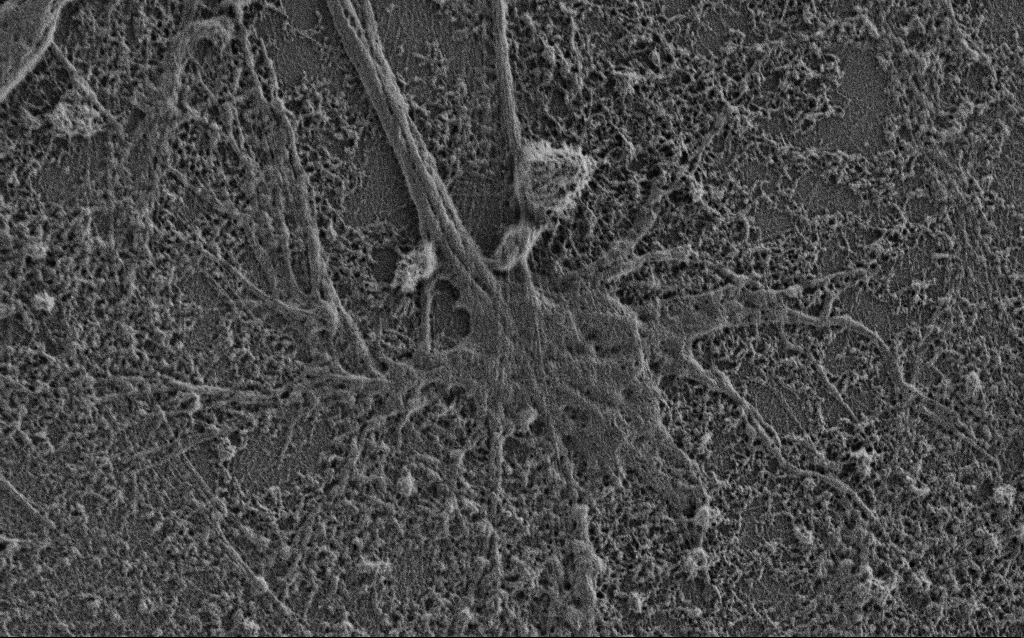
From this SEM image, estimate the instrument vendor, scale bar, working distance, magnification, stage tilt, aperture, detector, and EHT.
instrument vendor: Zeiss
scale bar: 2000 nm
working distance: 3.4 mm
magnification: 12.5 K X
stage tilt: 0°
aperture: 30 µm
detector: SE2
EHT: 0.9 kV